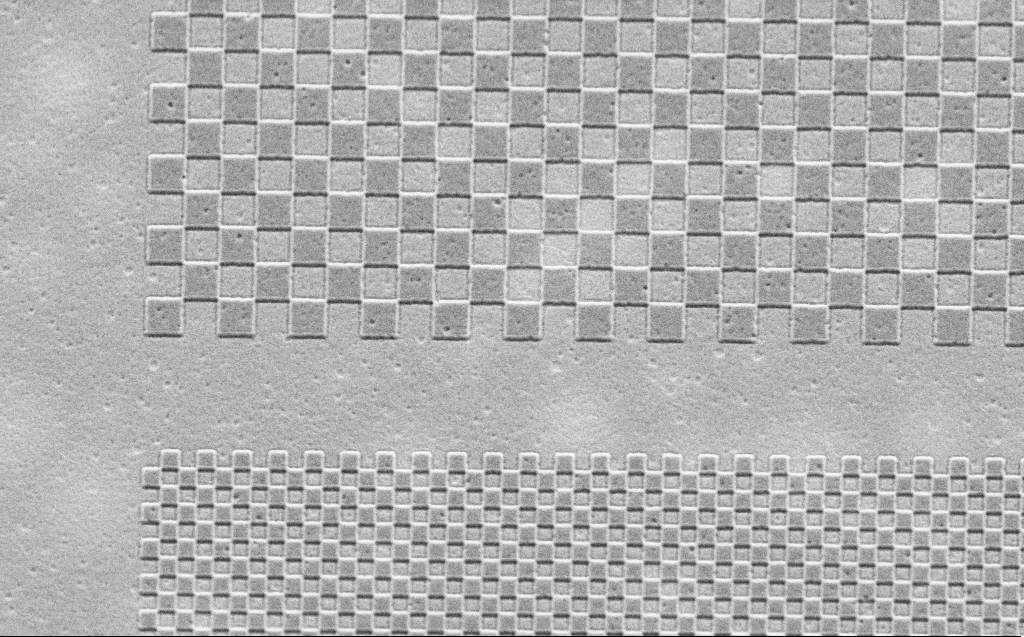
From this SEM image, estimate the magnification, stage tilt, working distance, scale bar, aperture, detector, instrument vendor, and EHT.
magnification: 13.34 K X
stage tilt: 30°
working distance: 4 mm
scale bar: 2000 nm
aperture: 30 µm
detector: SE2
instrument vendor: Zeiss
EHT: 2.5 kV